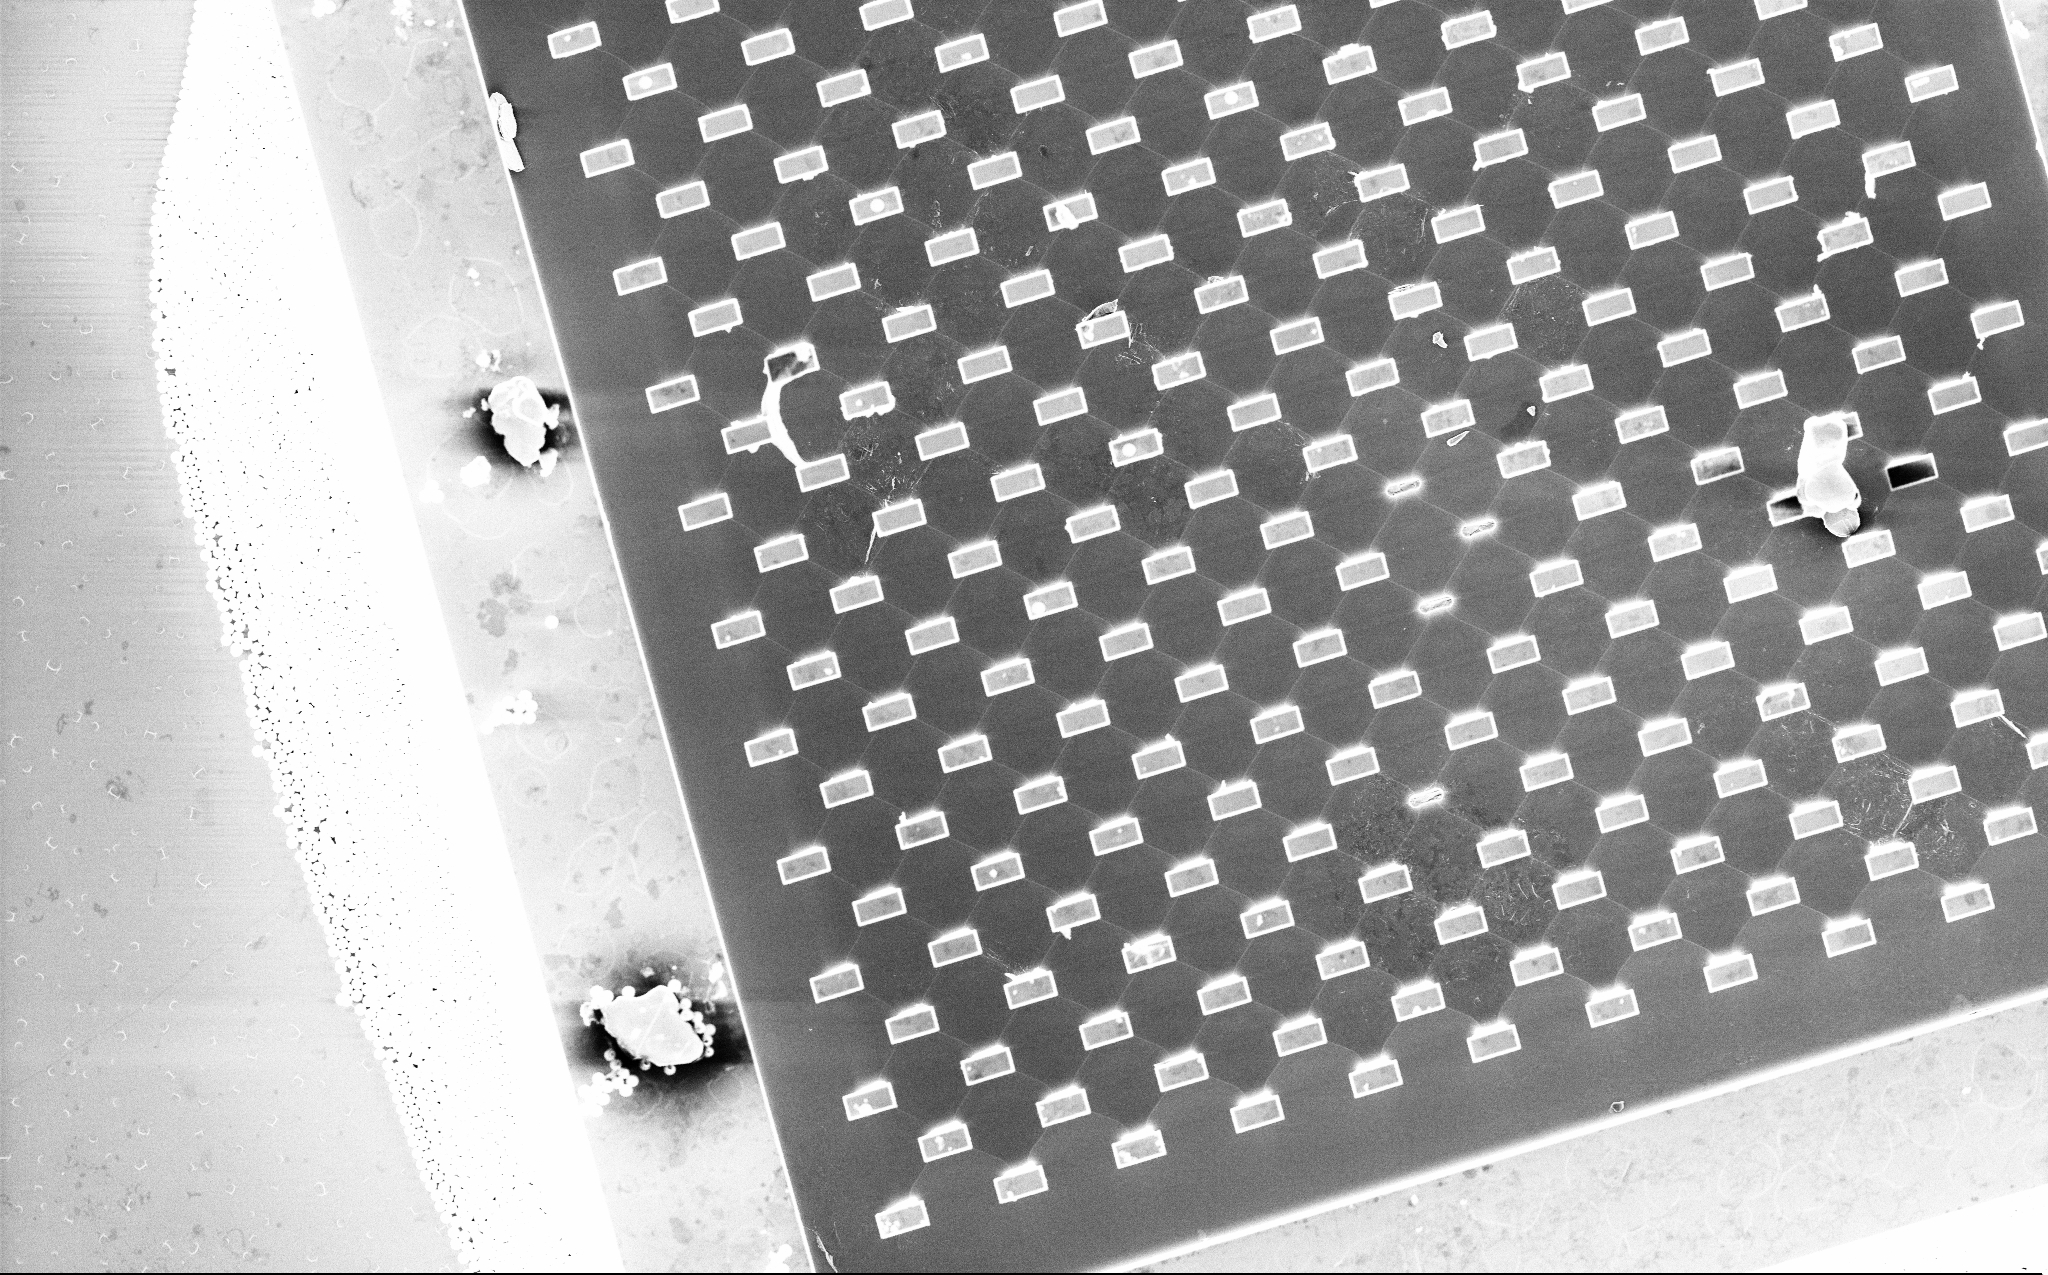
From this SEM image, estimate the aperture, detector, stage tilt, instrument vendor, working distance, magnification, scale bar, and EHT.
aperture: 30 µm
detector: InLens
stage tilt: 0°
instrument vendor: Zeiss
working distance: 4 mm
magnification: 1.13 K X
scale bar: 20000 nm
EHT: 5 kV